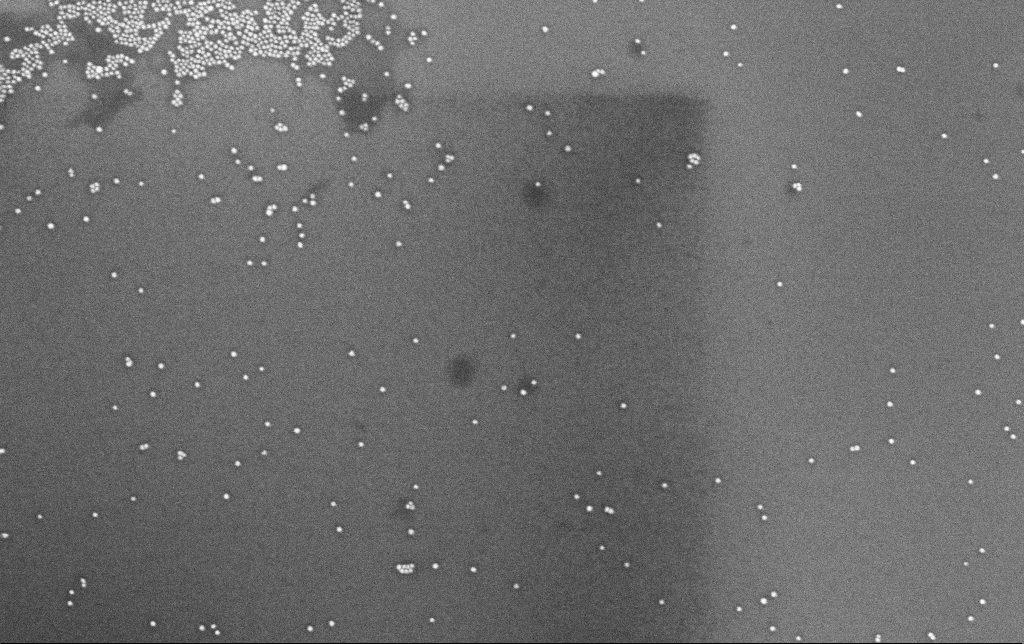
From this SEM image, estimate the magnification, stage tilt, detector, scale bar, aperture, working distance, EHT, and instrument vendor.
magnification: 100 K X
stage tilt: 0°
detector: InLens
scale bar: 200 nm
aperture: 30 µm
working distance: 6.6 mm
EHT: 8 kV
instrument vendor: Zeiss